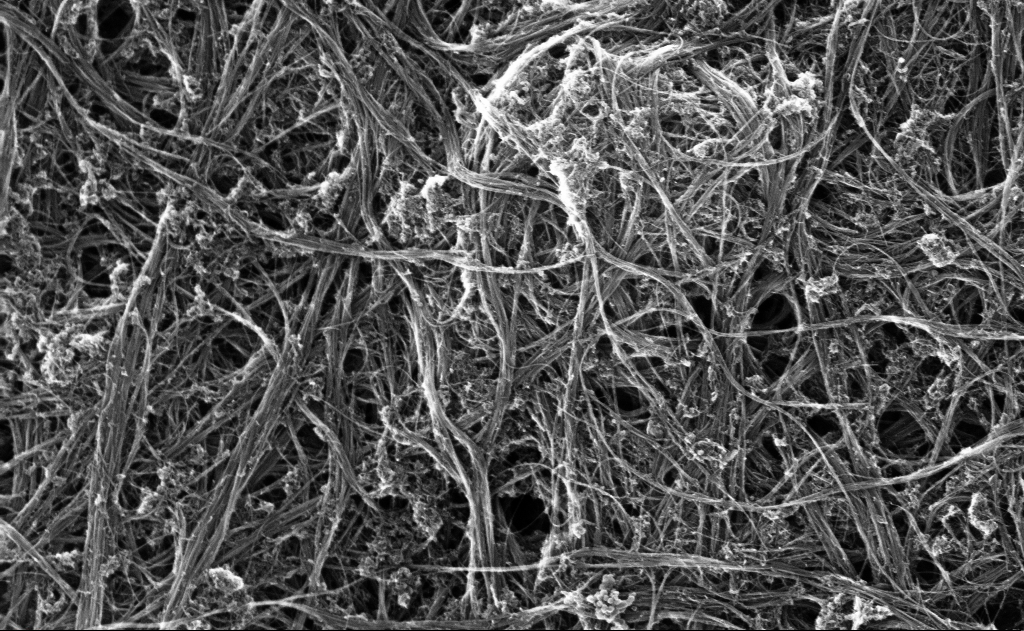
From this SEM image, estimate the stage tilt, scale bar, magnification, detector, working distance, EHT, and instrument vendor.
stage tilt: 0°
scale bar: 200 nm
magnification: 85.77 K X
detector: InLens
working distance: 5 mm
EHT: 10 kV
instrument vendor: Zeiss